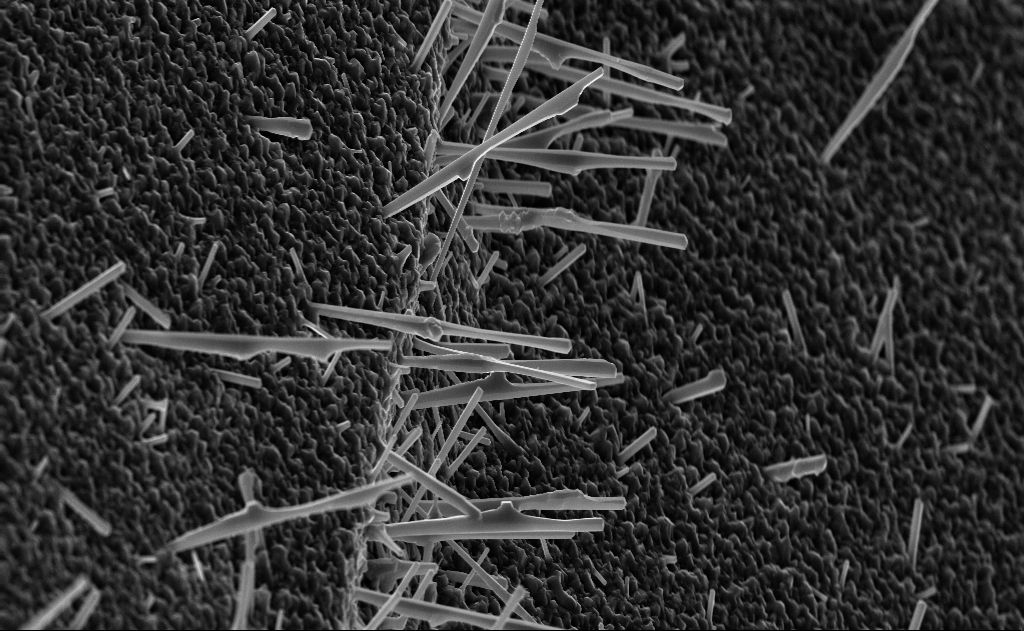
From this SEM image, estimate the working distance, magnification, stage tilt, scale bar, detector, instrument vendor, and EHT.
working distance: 5 mm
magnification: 10 K X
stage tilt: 0°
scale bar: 2000 nm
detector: InLens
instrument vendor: Zeiss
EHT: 10 kV